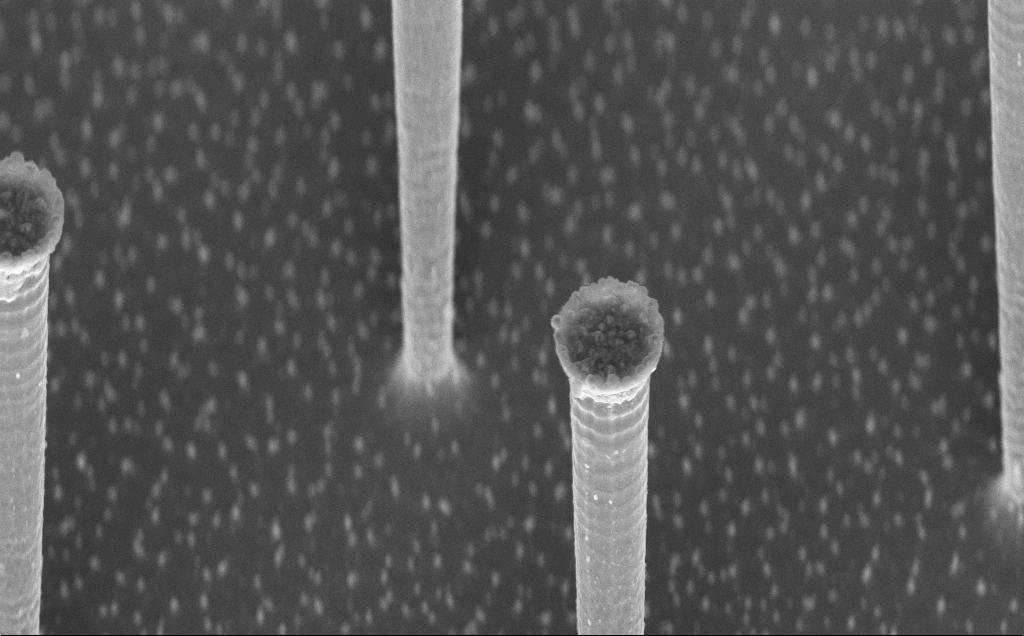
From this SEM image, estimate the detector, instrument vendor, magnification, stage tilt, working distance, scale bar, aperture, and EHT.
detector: InLens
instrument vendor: Zeiss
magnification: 9.07 K X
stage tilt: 44.9°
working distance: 7 mm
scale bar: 2000 nm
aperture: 30 µm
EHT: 7.5 kV